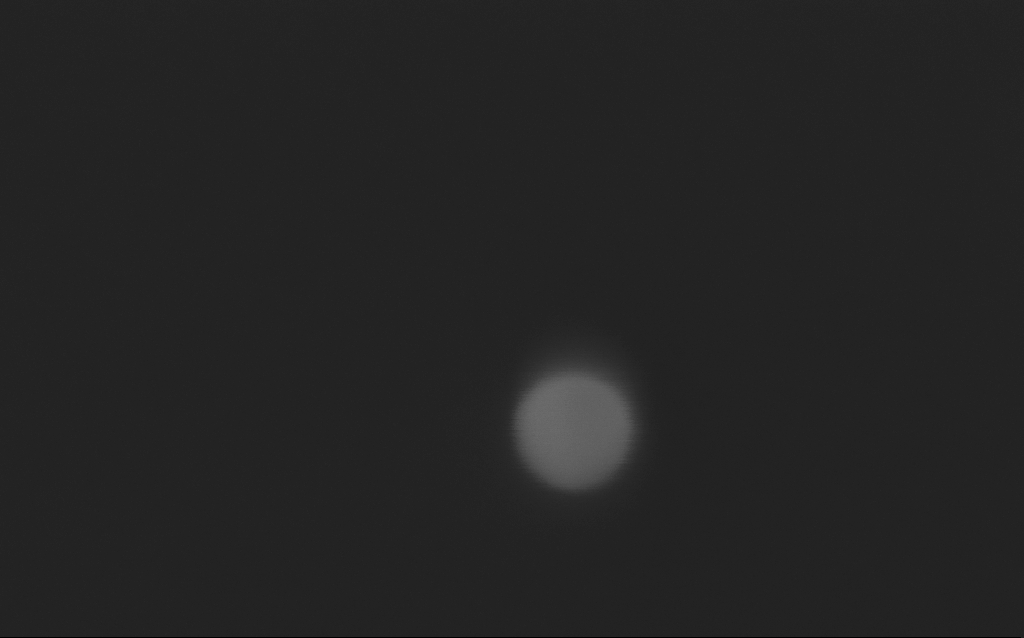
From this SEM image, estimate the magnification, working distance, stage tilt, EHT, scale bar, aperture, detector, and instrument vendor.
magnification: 833.68 K X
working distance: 3 mm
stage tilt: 0°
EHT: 5 kV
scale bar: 20 nm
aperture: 30 µm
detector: InLens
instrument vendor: Zeiss